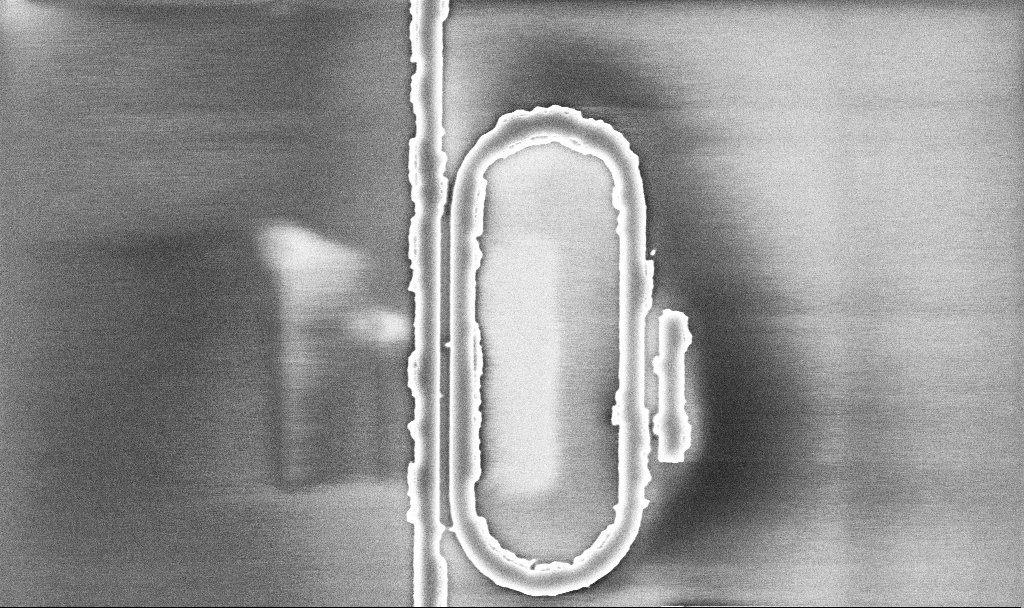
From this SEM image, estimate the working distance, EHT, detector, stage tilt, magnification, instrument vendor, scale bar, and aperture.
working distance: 10.1 mm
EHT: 5 kV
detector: InLens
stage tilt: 0°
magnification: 17.93 K X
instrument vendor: Zeiss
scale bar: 2000 nm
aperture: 30 µm